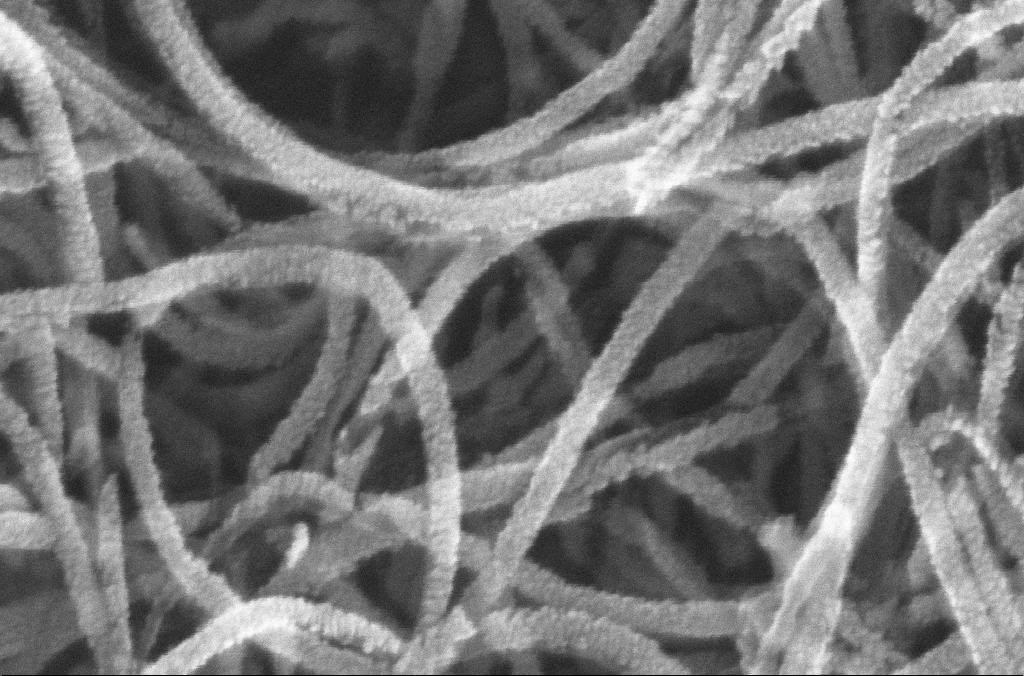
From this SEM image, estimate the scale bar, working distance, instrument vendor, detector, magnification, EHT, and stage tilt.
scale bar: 100 nm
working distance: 4.5 mm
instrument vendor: Zeiss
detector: InLens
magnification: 400 K X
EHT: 20 kV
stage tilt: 0°